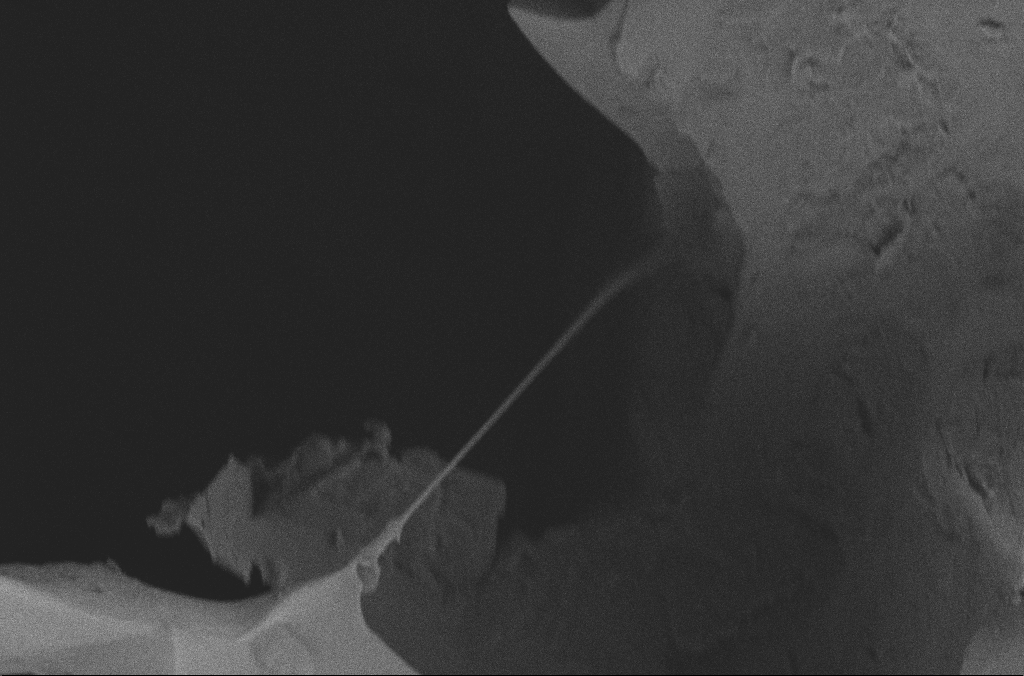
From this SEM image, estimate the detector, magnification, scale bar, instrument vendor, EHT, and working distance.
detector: SE2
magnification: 25 K X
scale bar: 2000 nm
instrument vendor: Zeiss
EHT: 2 kV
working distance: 3.1 mm